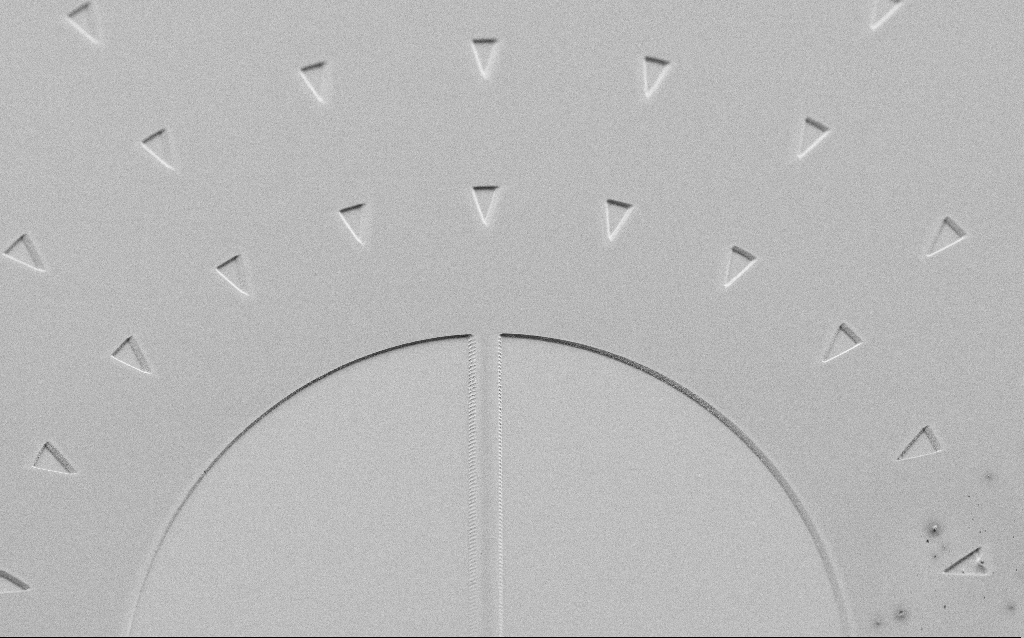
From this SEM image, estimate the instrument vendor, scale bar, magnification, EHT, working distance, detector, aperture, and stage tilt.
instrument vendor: Zeiss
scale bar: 100000 nm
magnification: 0.684 K X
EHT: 3 kV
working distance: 6 mm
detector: SE2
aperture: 30 µm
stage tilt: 45°